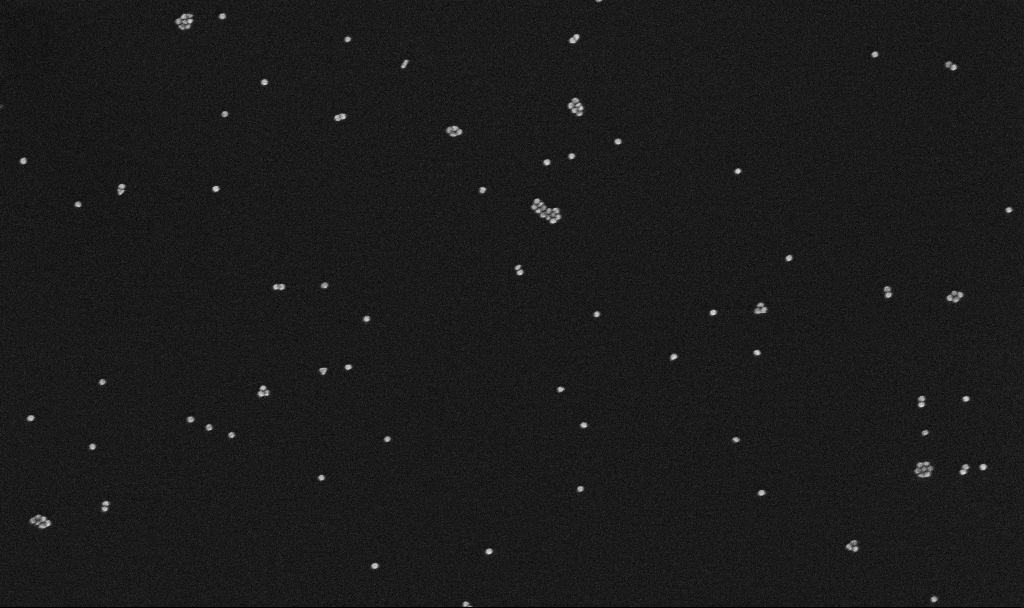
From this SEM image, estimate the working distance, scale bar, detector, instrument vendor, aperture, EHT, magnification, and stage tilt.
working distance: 3.3 mm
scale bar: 200 nm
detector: InLens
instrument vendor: Zeiss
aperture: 30 µm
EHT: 10 kV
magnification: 100 K X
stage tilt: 0°